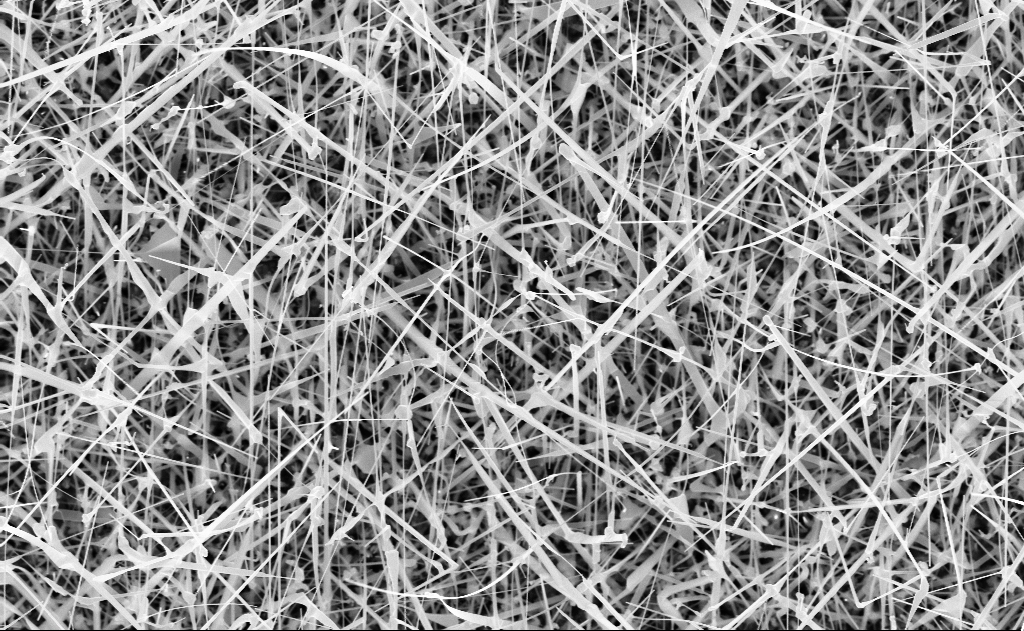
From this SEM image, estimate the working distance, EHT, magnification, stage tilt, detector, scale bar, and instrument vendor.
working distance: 10 mm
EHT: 10 kV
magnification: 20 K X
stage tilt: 0°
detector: InLens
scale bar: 1000 nm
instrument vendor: Zeiss